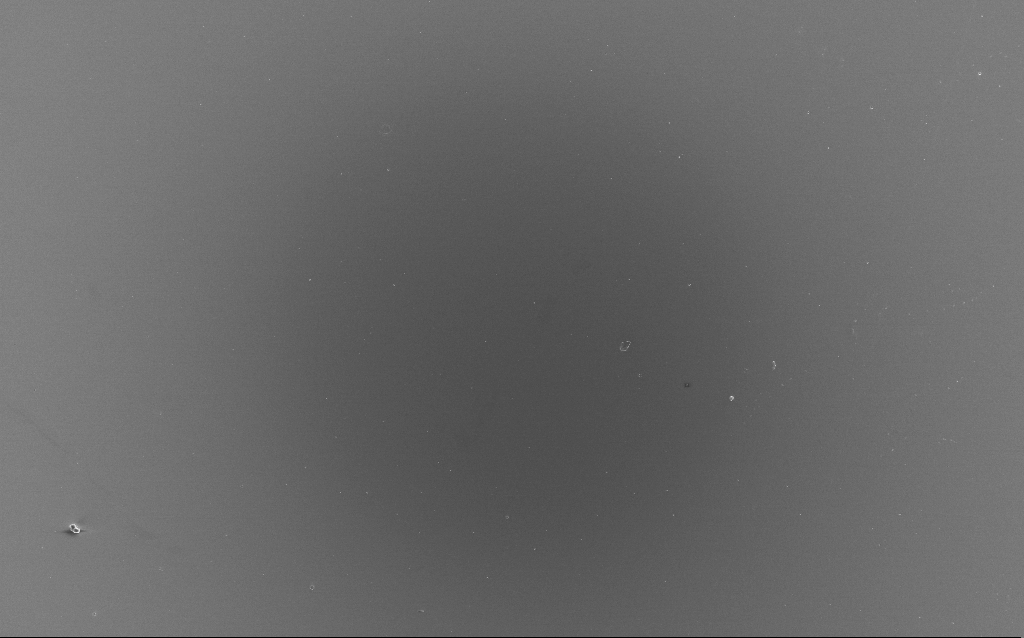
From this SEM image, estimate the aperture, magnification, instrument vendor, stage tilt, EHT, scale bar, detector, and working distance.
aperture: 30 µm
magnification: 0.442 K X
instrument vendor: Zeiss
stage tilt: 0°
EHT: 10 kV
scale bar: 100000 nm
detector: InLens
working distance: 2 mm